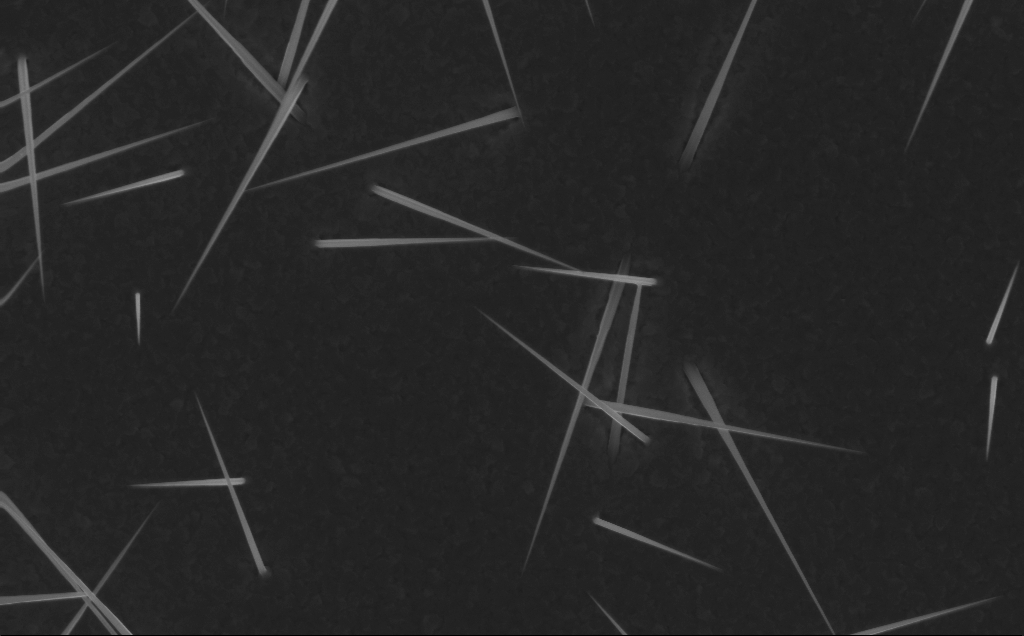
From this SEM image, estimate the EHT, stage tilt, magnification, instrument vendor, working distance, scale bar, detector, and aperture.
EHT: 10 kV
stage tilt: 0°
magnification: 20 K X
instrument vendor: Zeiss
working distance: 5 mm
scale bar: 1000 nm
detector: InLens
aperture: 30 µm